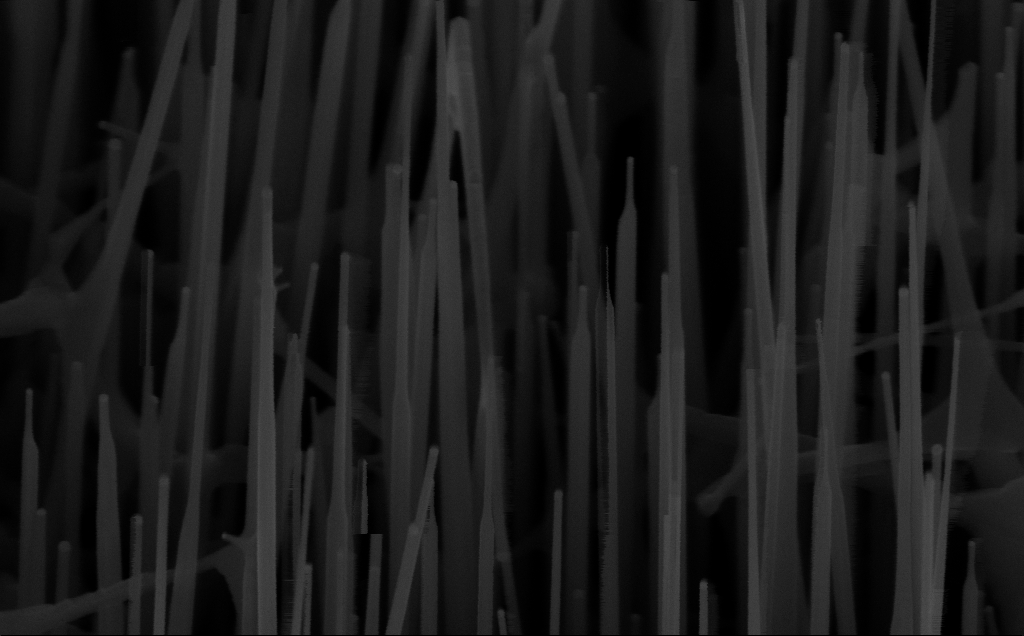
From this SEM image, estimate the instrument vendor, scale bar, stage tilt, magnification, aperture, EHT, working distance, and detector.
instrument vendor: Zeiss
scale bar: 200 nm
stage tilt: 45°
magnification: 150 K X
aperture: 30 µm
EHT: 10 kV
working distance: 7 mm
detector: InLens